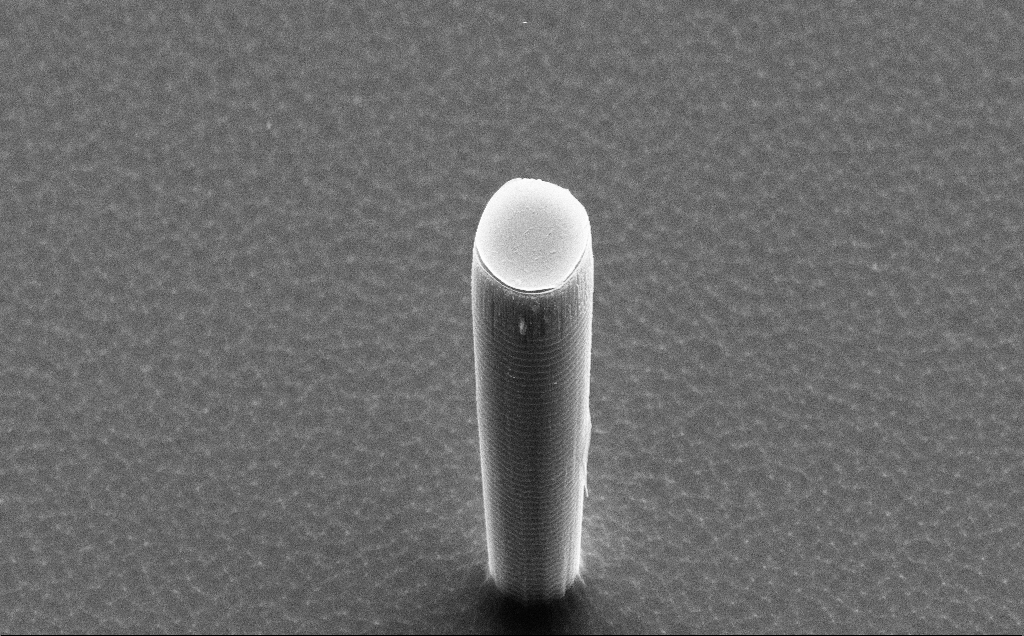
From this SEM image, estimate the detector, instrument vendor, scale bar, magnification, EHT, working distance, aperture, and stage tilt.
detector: SE2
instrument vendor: Zeiss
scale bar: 10000 nm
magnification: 6.45 K X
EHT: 15 kV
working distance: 10 mm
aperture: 30 µm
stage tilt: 50°